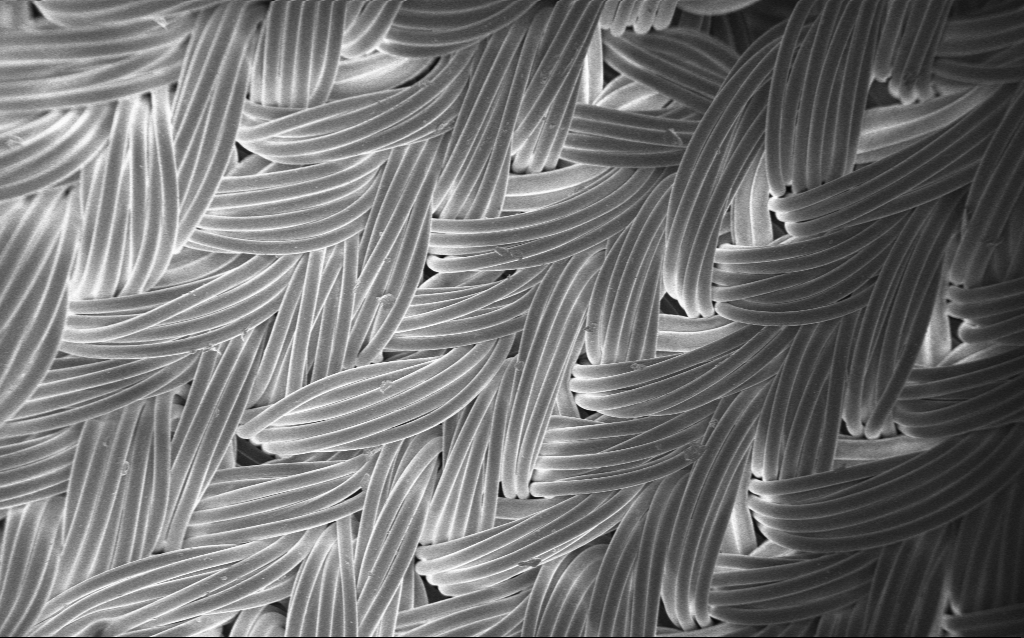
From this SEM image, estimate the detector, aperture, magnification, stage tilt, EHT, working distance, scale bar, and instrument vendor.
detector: InLens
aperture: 30 µm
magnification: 0.225 K X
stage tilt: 0°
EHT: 1 kV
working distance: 4 mm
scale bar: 100000 nm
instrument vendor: Zeiss